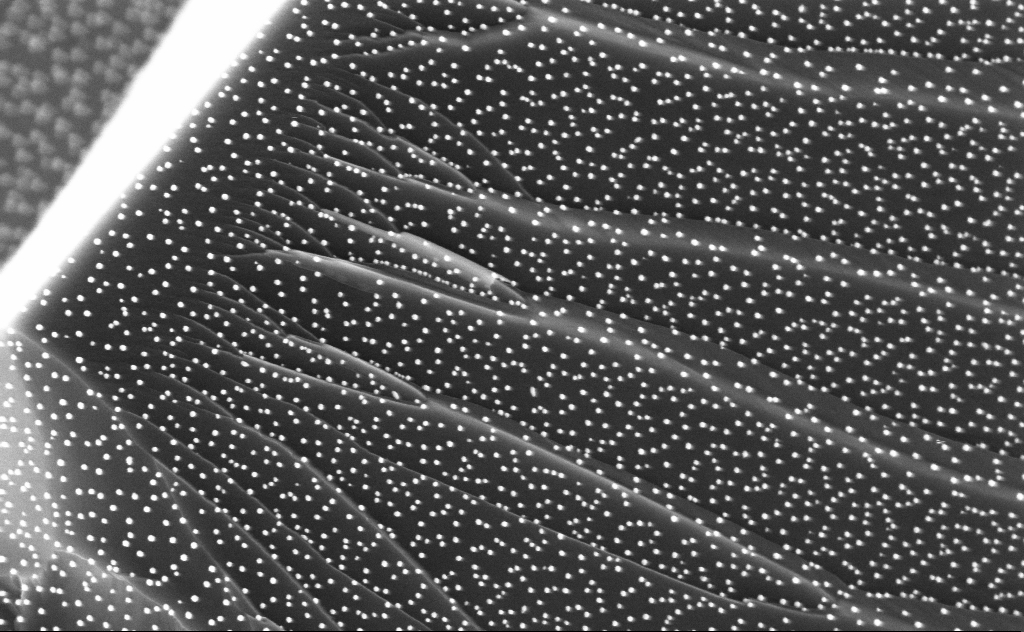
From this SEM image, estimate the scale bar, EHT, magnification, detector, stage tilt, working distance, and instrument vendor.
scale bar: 200 nm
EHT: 3 kV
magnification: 100 K X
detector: InLens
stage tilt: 0°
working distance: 5 mm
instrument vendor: Zeiss